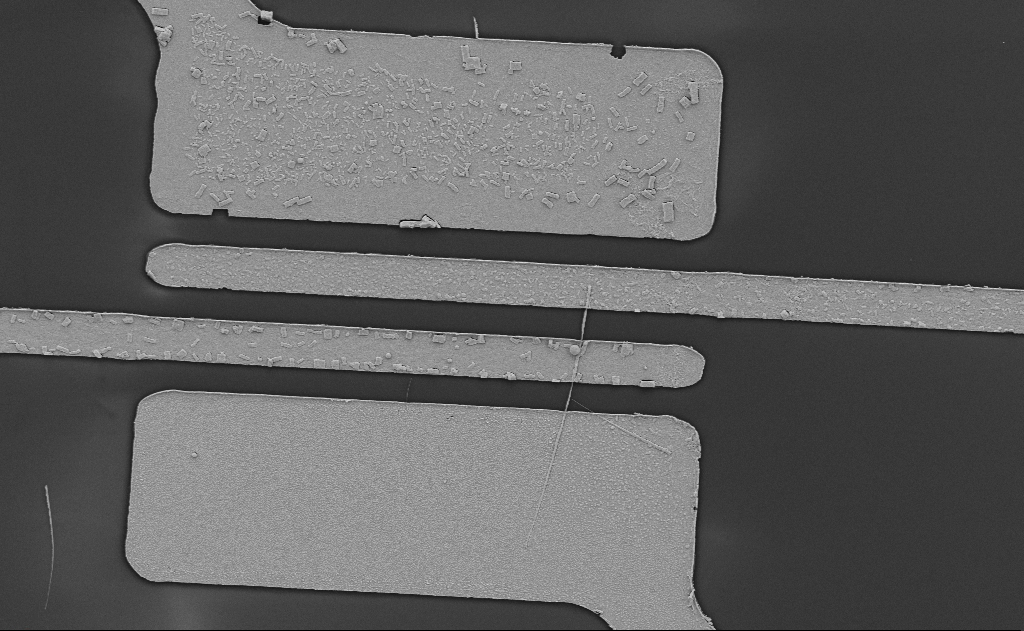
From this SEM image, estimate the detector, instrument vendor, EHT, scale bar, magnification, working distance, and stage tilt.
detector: SE2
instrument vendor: Zeiss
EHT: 5 kV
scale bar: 10000 nm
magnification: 6.75 K X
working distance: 15 mm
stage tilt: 0°